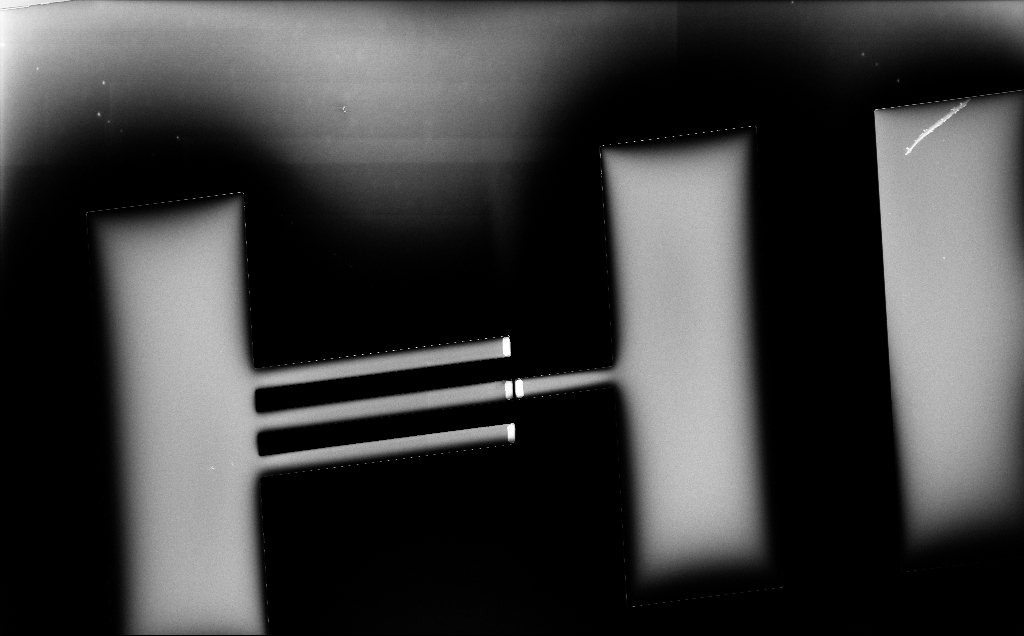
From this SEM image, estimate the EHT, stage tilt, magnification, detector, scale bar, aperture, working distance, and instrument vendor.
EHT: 5 kV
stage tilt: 0°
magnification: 0.293 K X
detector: InLens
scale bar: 100000 nm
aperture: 30 µm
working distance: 6 mm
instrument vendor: Zeiss